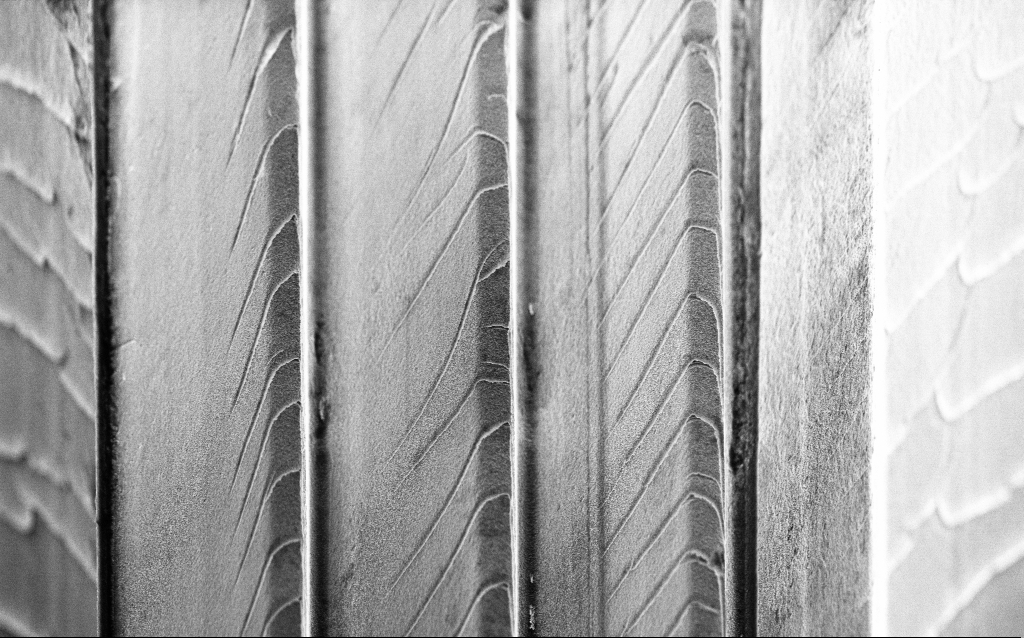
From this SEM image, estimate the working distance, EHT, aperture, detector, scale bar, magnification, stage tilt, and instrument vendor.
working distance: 7 mm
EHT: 5 kV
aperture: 30 µm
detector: InLens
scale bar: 10000 nm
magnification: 6.84 K X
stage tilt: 45°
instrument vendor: Zeiss